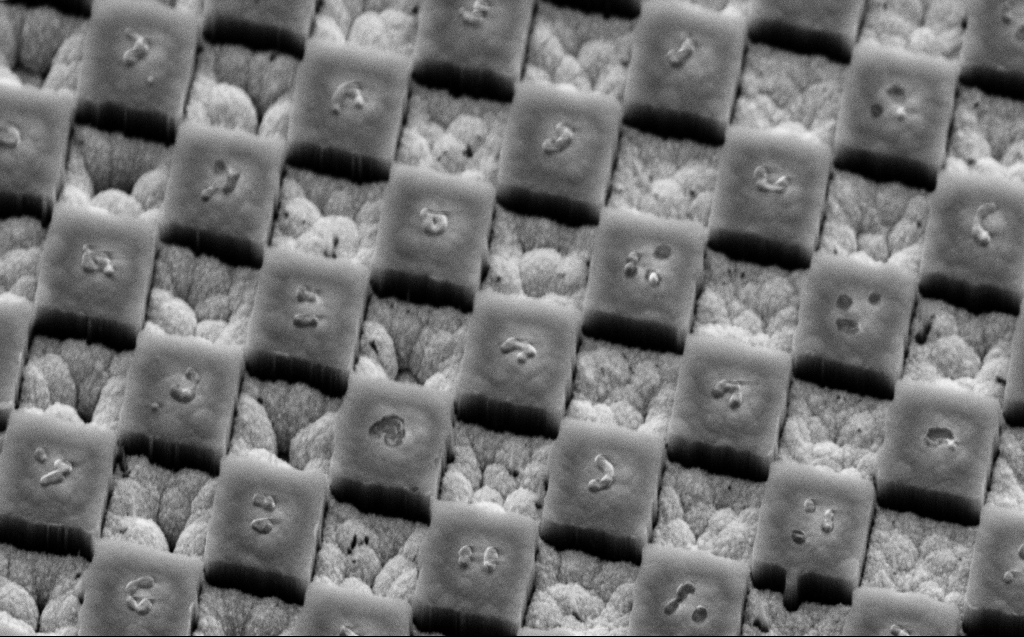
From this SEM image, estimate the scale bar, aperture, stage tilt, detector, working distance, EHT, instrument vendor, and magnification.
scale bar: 1000 nm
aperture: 30 µm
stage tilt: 45°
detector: SE2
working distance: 9 mm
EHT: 5 kV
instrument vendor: Zeiss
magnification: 39.5 K X